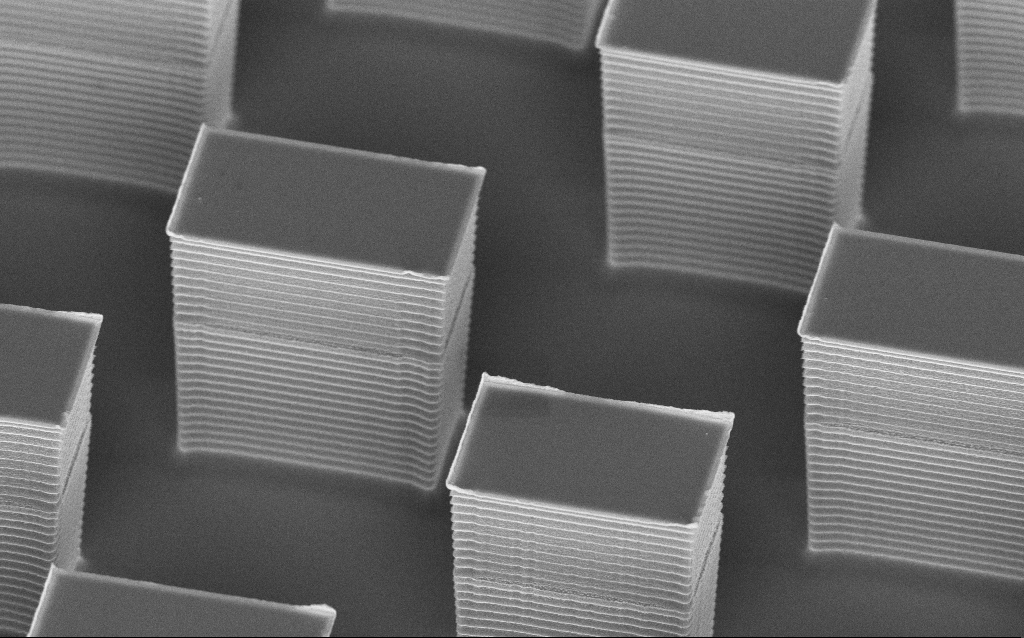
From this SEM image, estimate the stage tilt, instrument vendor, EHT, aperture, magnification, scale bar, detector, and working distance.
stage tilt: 45°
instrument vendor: Zeiss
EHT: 5 kV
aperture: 30 µm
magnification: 11.83 K X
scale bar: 2000 nm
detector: InLens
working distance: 8 mm